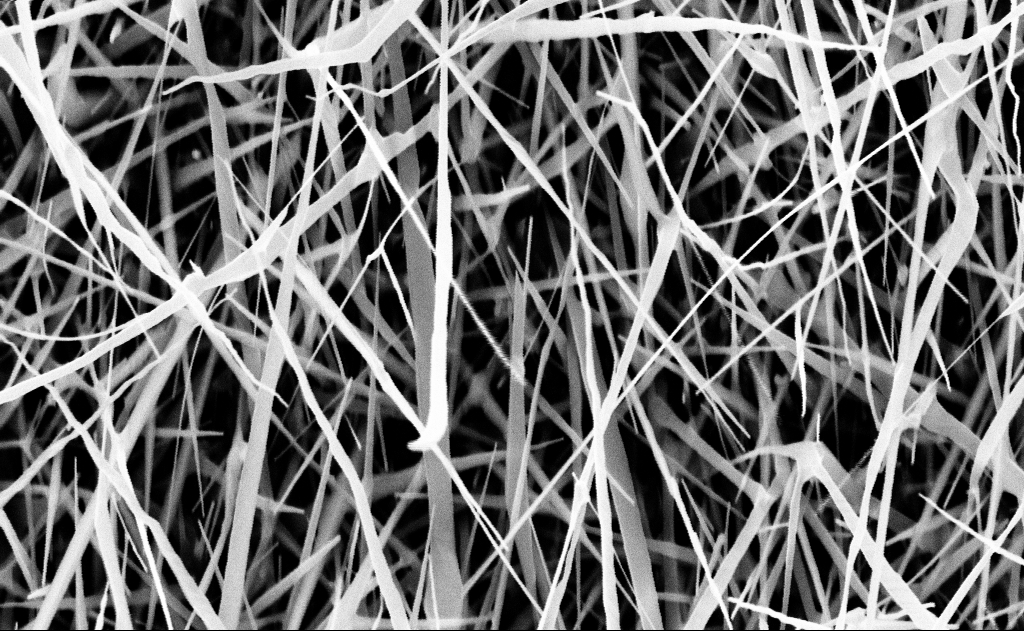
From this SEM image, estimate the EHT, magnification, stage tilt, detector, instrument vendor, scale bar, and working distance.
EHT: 10 kV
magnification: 40 K X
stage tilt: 0°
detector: InLens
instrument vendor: Zeiss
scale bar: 1000 nm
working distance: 17 mm